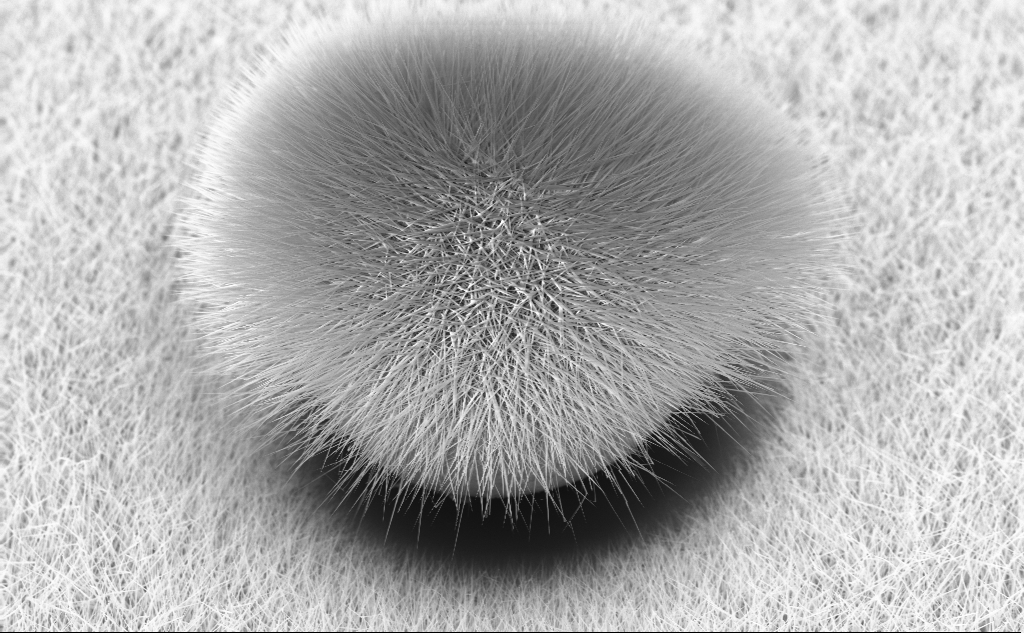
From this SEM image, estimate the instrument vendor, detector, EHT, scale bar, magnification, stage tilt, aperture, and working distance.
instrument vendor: Zeiss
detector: InLens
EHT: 10 kV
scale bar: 10000 nm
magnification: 6.65 K X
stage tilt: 45°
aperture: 30 µm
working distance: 4 mm